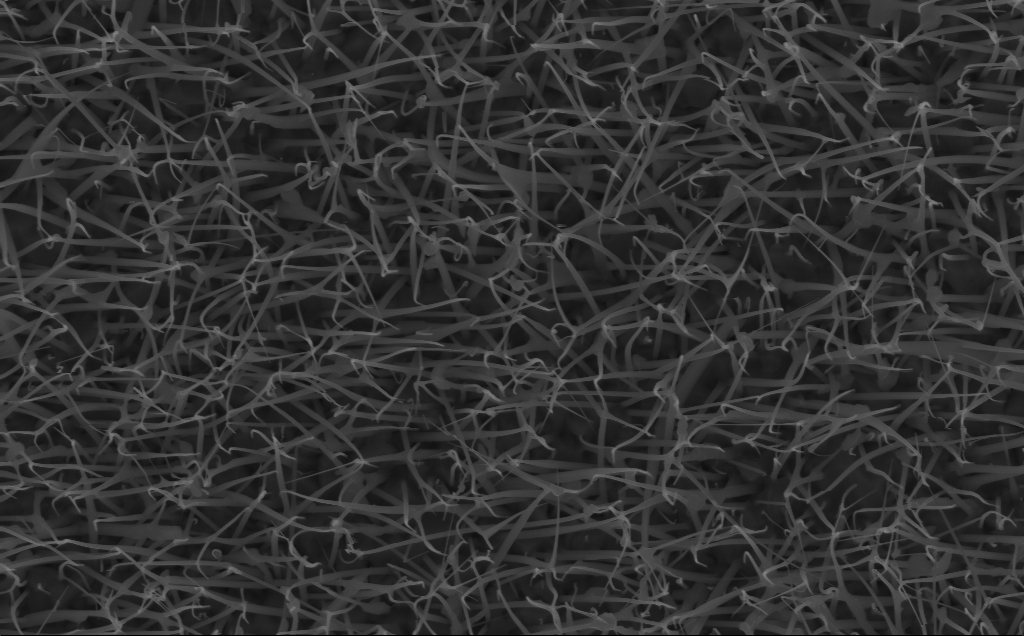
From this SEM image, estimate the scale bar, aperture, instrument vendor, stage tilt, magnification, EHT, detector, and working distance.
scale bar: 1000 nm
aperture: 30 µm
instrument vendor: Zeiss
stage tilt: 0°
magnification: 20 K X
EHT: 10 kV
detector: InLens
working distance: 6 mm